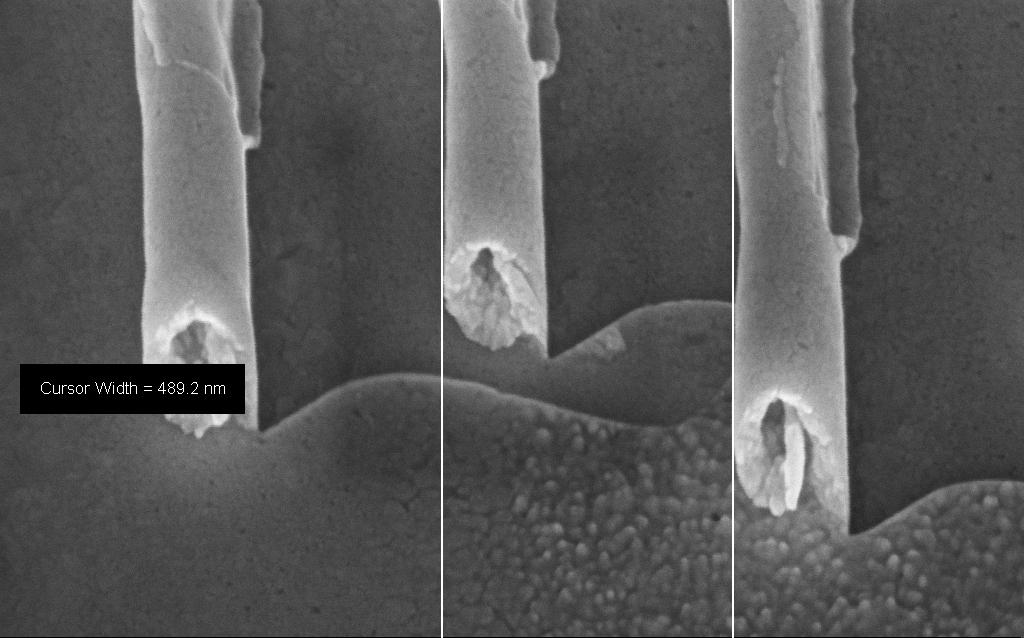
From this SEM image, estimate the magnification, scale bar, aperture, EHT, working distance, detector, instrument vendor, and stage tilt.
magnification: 218.43 K X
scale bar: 200 nm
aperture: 30 µm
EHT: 10 kV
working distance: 7 mm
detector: InLens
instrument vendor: Zeiss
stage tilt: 45°